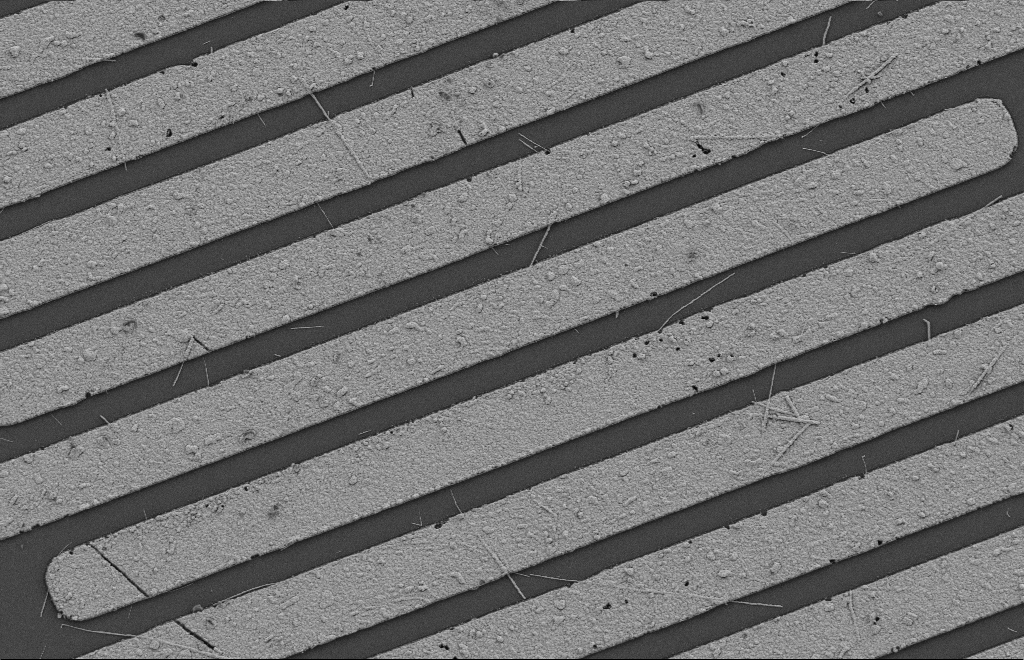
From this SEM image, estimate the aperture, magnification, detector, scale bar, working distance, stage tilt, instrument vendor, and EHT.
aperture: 20 µm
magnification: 6.36 K X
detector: SE2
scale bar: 2000 nm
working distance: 8 mm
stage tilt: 0°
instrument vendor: Zeiss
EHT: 2 kV